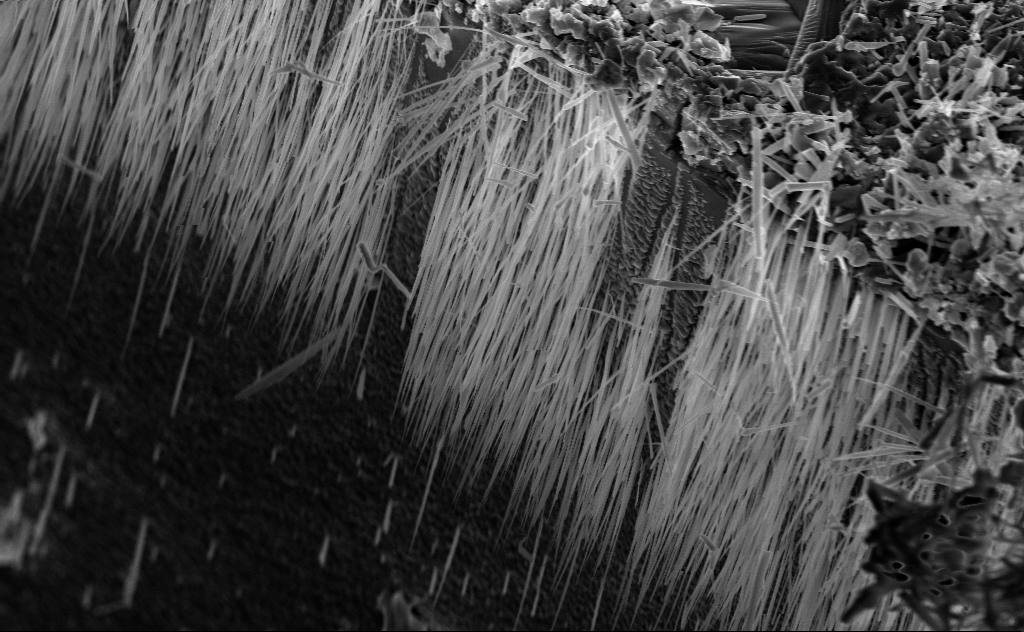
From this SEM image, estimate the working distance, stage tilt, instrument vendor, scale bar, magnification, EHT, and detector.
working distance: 7 mm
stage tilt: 45°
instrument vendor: Zeiss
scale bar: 2000 nm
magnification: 11.07 K X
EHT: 10 kV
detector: InLens